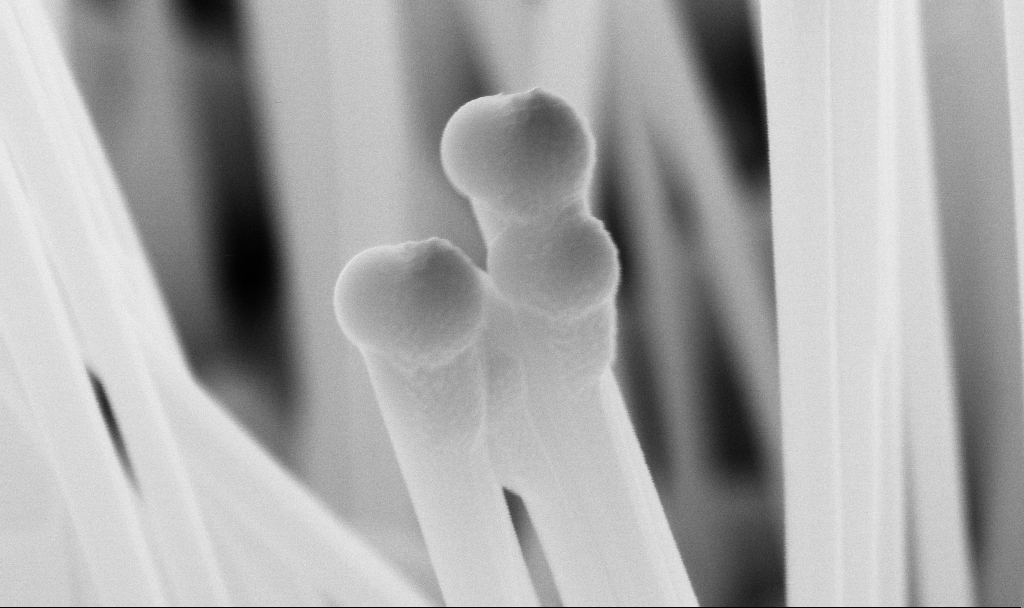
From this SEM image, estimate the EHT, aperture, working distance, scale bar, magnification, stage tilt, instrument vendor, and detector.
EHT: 10 kV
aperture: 30 µm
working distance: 7.1 mm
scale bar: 200 nm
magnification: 200 K X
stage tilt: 45°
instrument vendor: Zeiss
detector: InLens